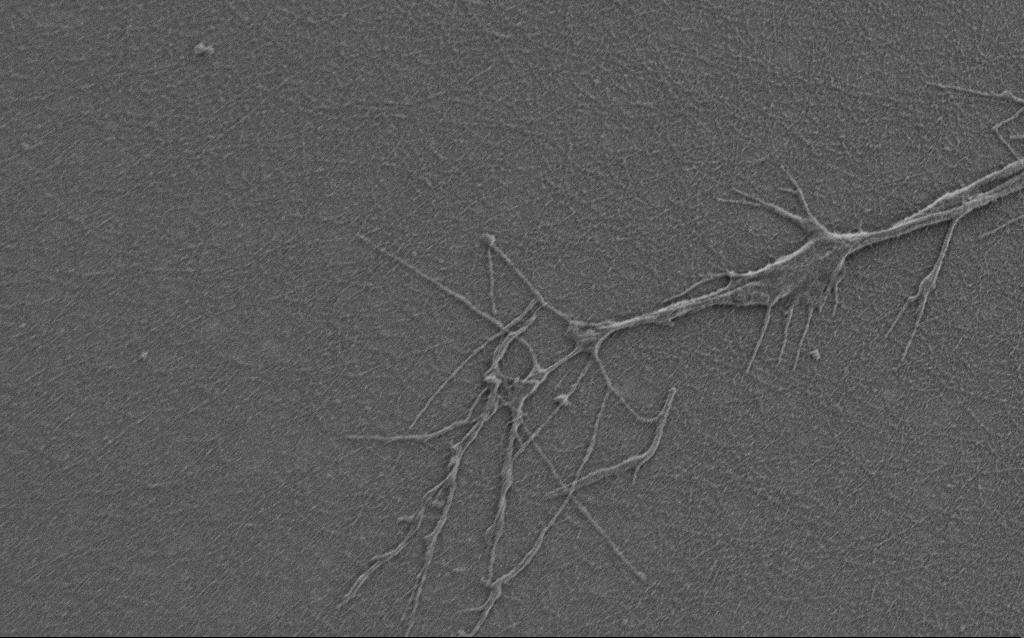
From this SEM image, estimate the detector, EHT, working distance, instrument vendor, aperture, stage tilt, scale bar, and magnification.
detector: SE2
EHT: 0.9 kV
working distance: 6 mm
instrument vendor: Zeiss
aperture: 30 µm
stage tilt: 0°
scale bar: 2000 nm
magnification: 10 K X